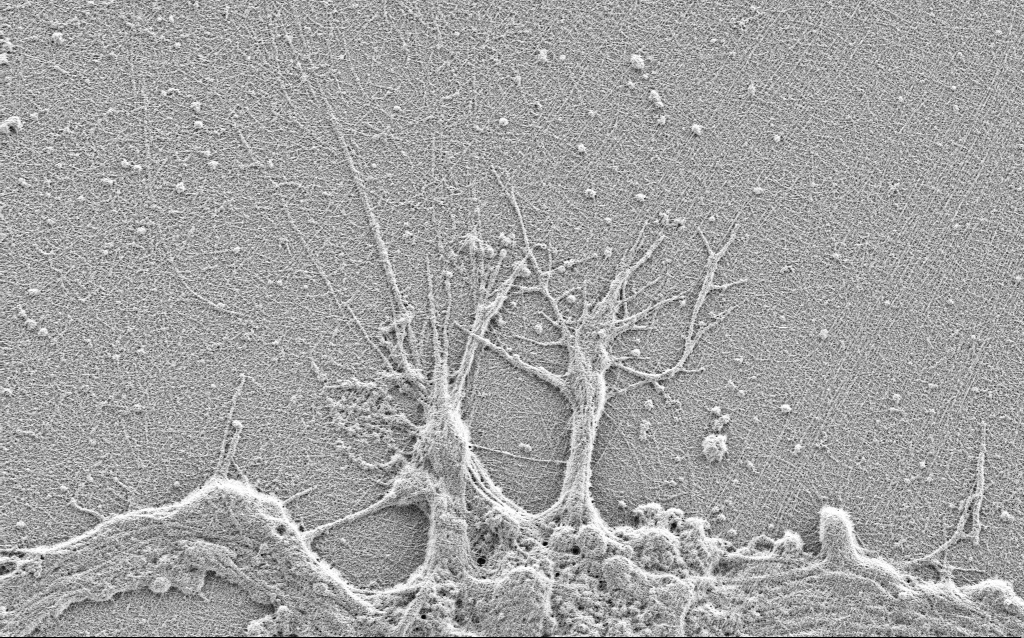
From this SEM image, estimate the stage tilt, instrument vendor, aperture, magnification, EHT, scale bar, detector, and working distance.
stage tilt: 0°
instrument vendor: Zeiss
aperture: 30 µm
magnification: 7.5 K X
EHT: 0.9 kV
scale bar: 2000 nm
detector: SE2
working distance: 3 mm